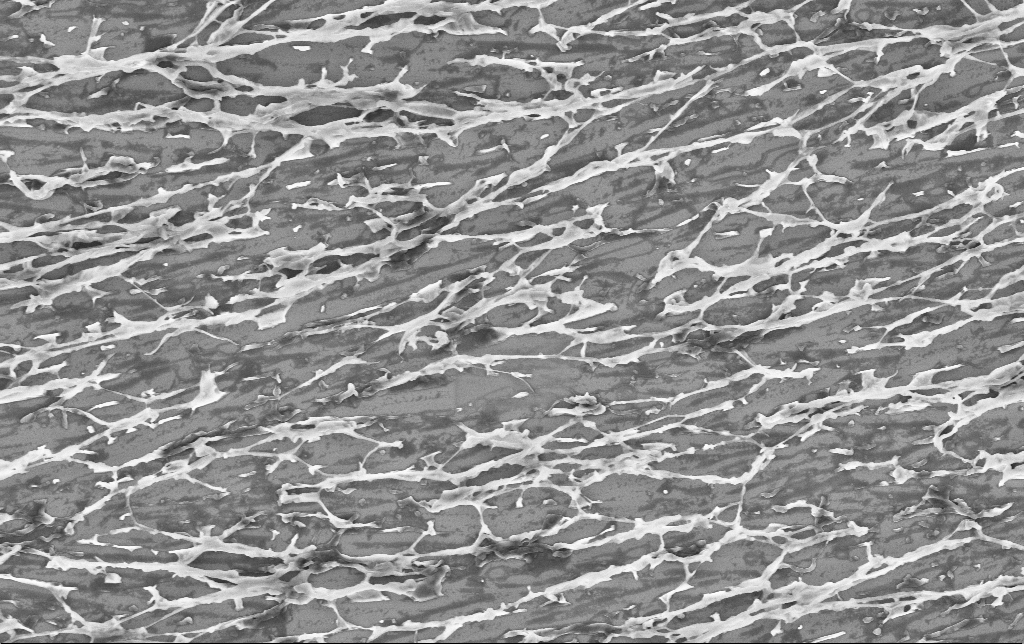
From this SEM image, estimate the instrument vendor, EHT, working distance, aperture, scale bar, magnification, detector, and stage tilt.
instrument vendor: Zeiss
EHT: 3 kV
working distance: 3.4 mm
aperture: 30 µm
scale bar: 2000 nm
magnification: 12.44 K X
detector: InLens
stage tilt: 0°